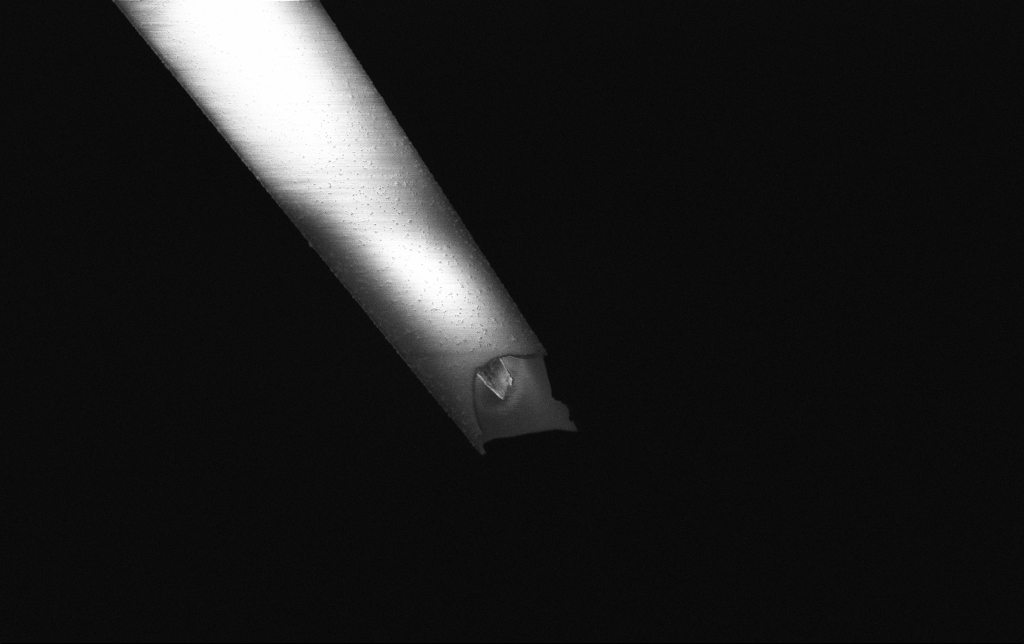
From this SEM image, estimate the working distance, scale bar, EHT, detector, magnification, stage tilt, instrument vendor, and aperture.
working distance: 6.7 mm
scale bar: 2000 nm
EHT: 3 kV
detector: InLens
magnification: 10 K X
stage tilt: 0°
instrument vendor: Zeiss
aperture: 30 µm